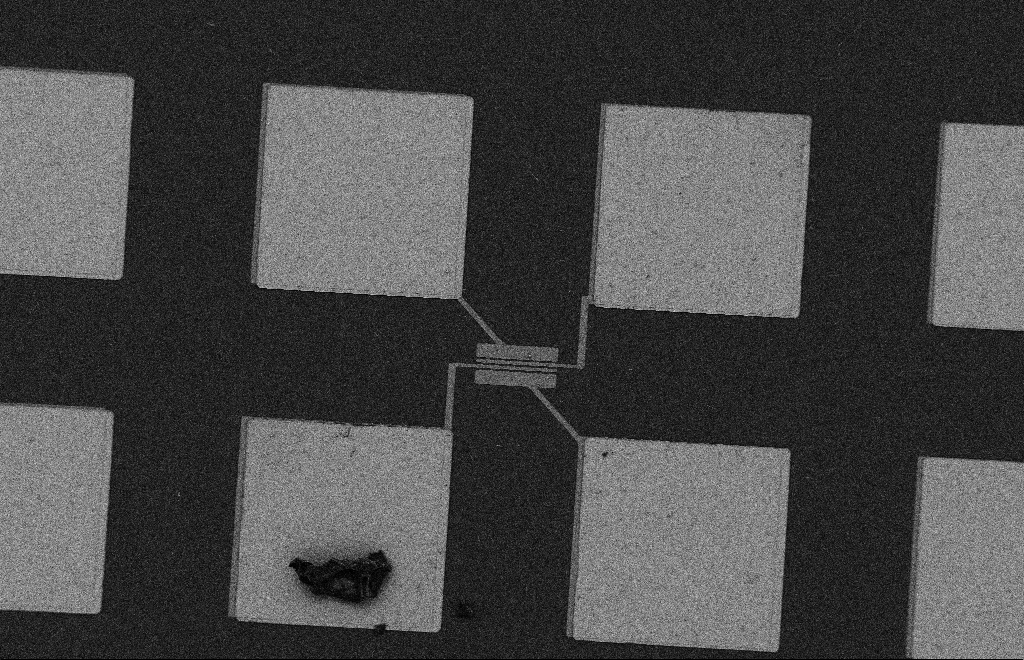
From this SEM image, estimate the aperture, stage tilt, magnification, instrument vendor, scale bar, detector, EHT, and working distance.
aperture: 20 µm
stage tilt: -0.3°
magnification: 0.489 K X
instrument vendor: Zeiss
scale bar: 20000 nm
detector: SE2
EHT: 2 kV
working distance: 10 mm